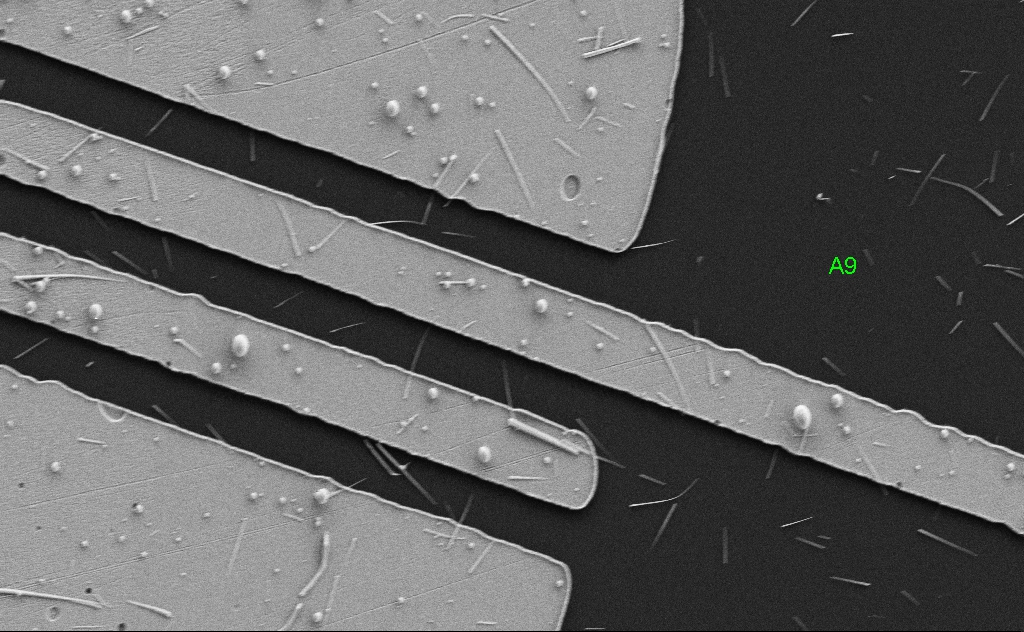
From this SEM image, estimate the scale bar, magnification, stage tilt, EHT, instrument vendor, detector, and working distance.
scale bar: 2000 nm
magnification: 8.87 K X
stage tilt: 0°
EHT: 5 kV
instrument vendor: Zeiss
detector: SE2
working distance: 5 mm